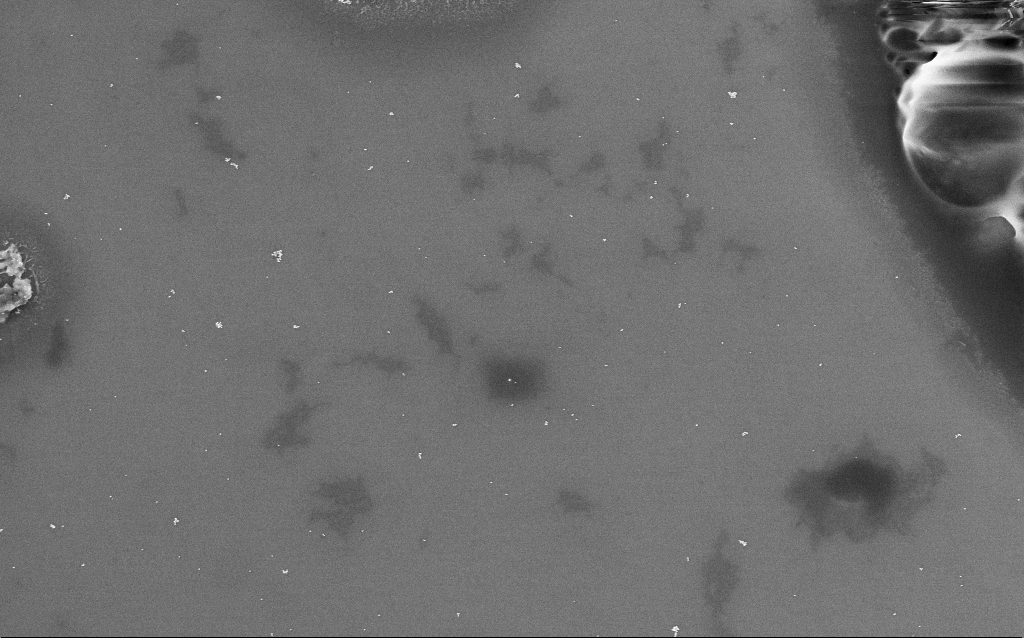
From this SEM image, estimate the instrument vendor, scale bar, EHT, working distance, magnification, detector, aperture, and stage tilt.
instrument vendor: Zeiss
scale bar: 1000 nm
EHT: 4 kV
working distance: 2.9 mm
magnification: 29.63 K X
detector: InLens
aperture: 30 µm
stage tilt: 0°